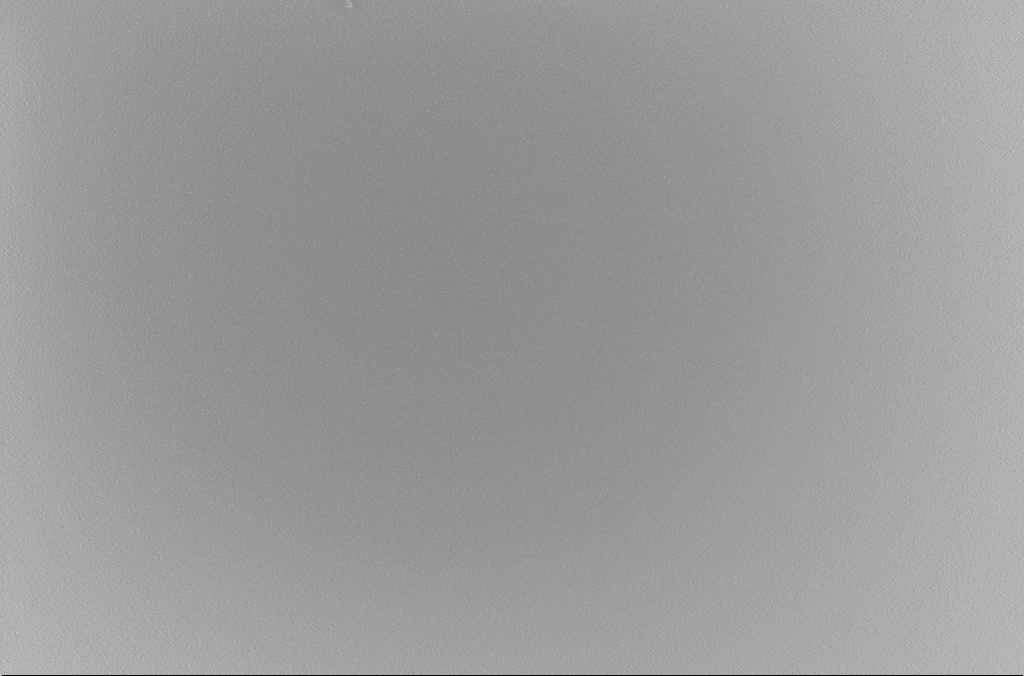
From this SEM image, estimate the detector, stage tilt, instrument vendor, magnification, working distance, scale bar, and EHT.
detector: InLens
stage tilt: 0°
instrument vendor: Zeiss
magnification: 1 K X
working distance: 3 mm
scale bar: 20000 nm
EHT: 5 kV